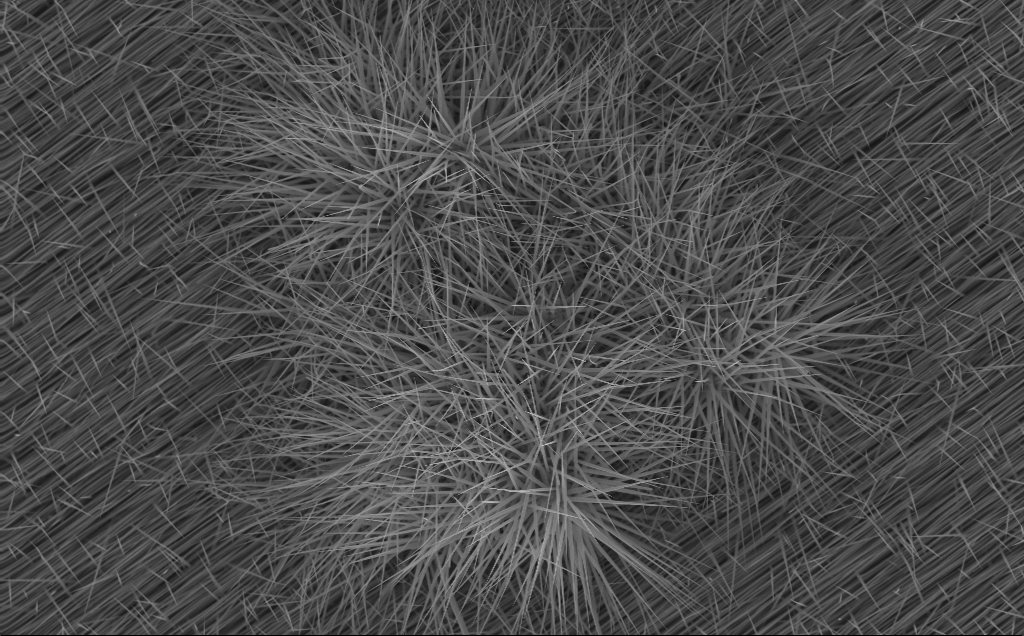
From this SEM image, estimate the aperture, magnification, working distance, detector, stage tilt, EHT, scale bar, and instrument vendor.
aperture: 30 µm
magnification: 11 K X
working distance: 6 mm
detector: InLens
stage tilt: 0°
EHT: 10 kV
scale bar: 2000 nm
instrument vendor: Zeiss